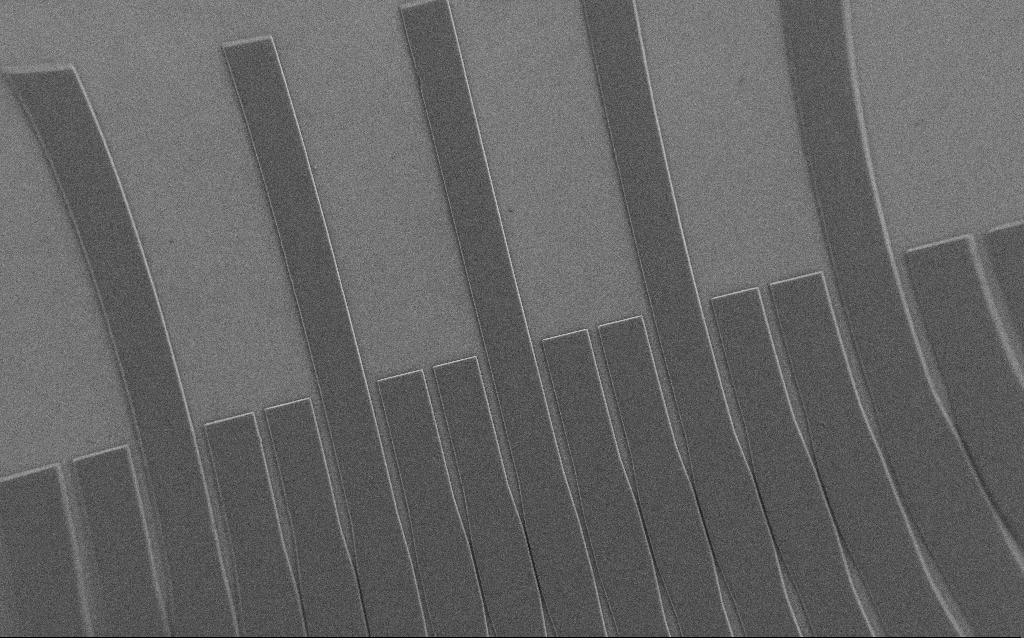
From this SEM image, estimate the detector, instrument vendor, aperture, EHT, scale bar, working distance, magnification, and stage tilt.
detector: SE2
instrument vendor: Zeiss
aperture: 30 µm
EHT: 1 kV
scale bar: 100000 nm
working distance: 6 mm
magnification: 0.195 K X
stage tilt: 0°